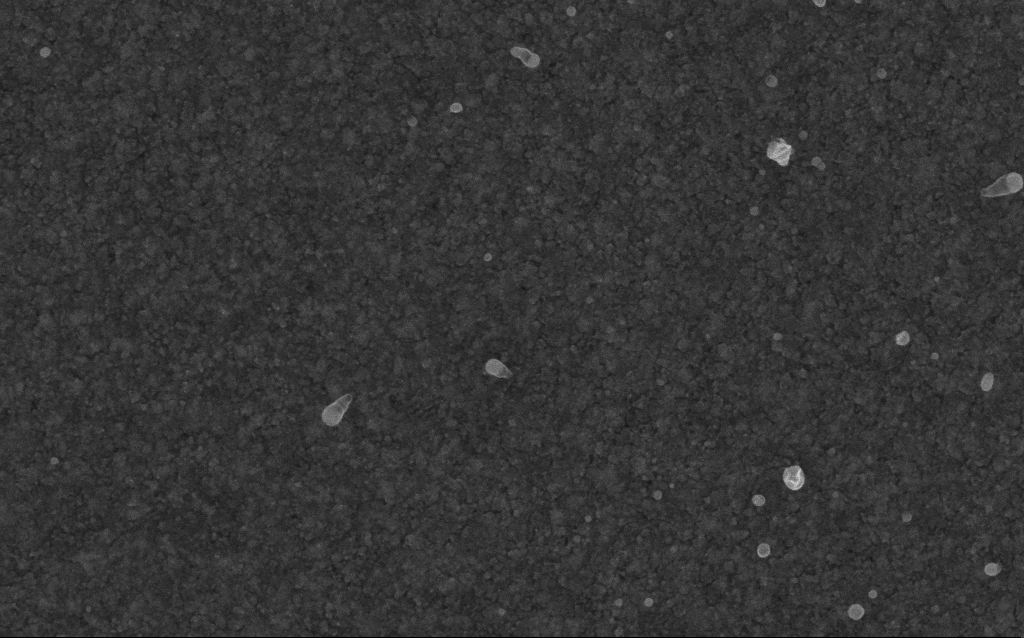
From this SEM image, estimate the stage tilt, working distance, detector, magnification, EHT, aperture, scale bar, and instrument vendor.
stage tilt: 0°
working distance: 2.6 mm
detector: InLens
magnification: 121.4 K X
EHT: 5 kV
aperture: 30 µm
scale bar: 200 nm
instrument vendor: Zeiss